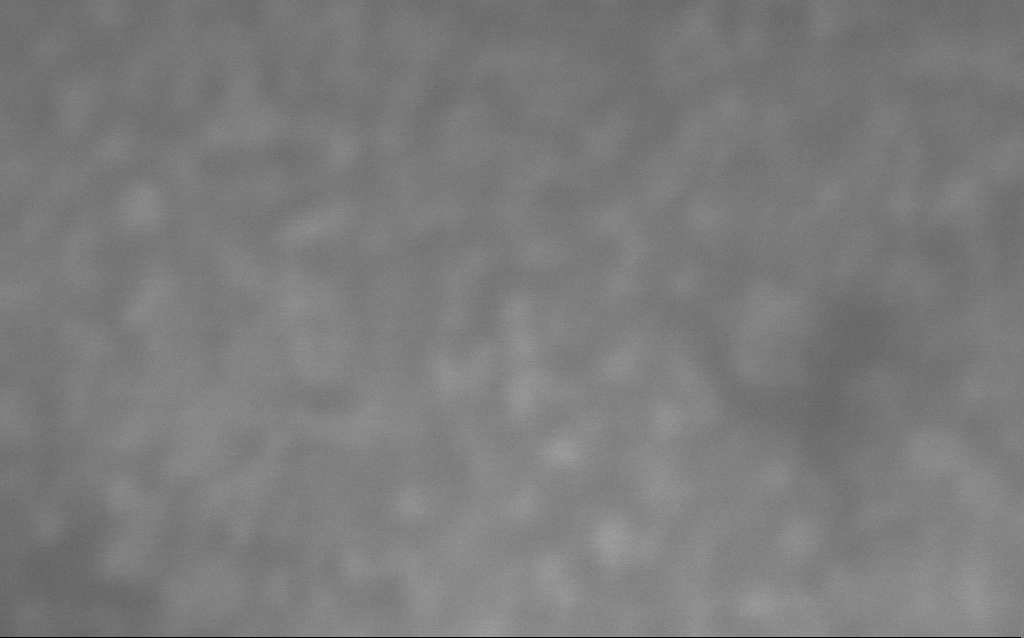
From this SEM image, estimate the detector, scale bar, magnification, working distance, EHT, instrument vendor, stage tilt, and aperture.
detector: InLens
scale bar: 20 nm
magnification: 2496.81 K X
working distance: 2 mm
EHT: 10 kV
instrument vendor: Zeiss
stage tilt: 0°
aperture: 30 µm